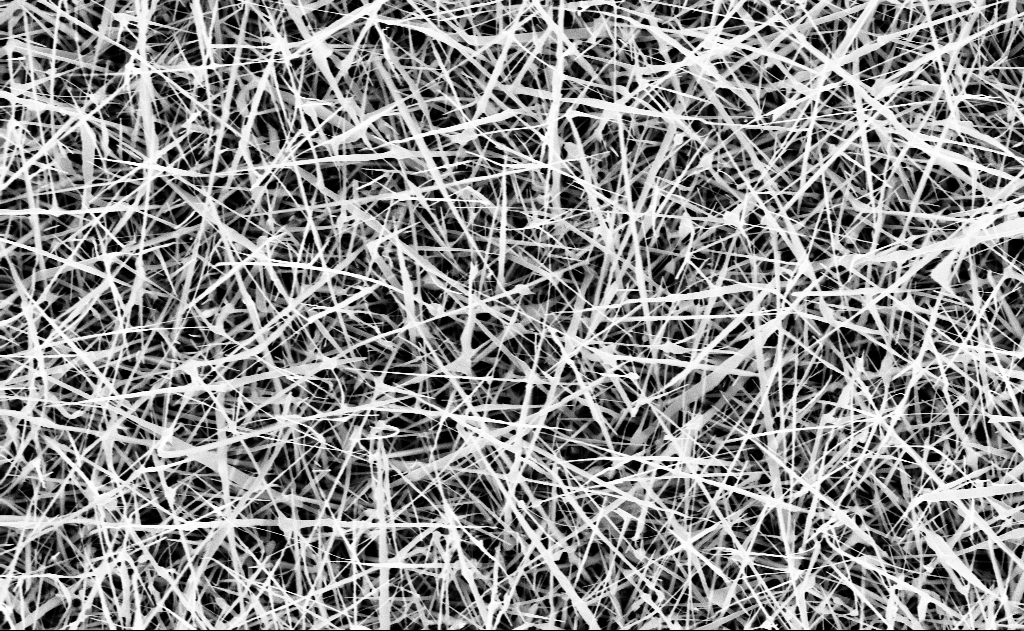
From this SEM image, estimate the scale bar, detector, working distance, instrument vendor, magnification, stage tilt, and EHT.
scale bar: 2000 nm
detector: InLens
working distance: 15 mm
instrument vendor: Zeiss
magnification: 20 K X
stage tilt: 0°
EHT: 10 kV